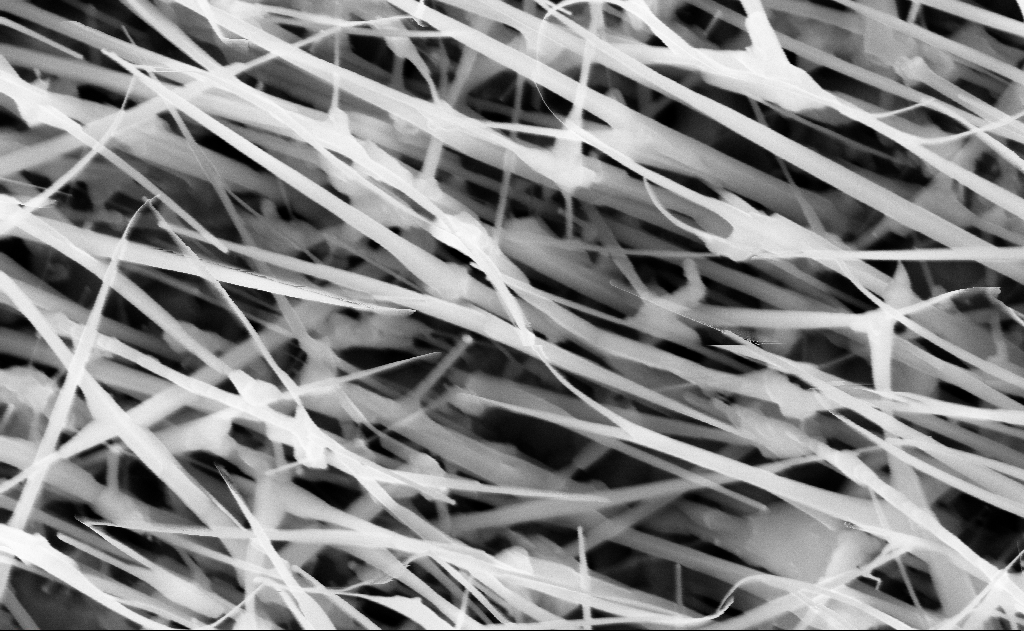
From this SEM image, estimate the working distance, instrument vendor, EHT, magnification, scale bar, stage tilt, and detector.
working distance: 13 mm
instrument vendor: Zeiss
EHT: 10 kV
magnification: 80 K X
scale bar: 200 nm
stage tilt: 0°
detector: InLens